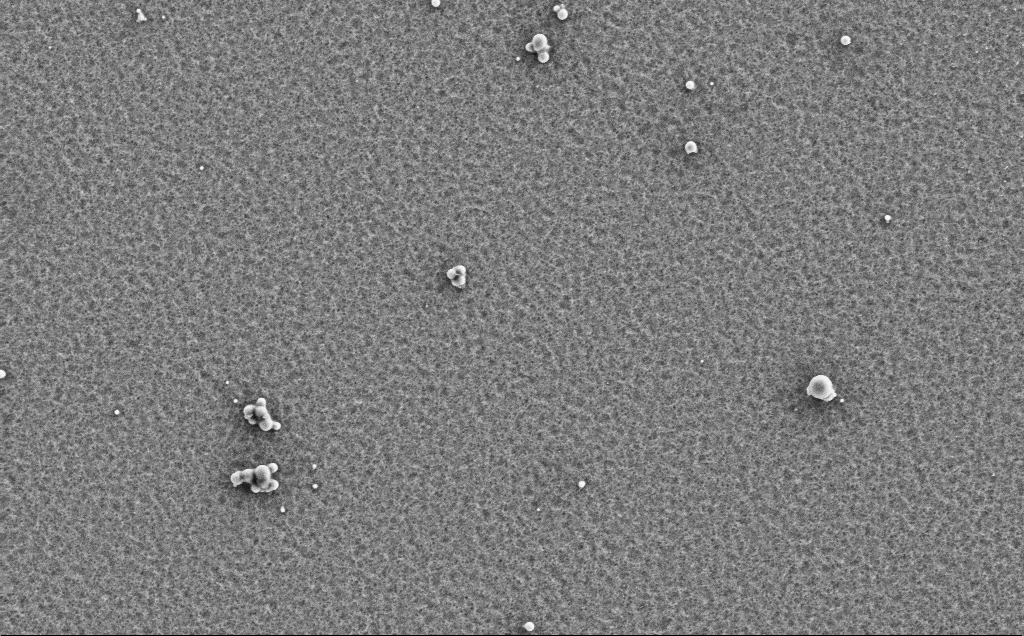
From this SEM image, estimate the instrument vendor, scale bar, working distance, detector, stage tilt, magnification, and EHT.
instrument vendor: Zeiss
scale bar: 10000 nm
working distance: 5 mm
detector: SE2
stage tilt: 0°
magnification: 4.14 K X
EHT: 5 kV